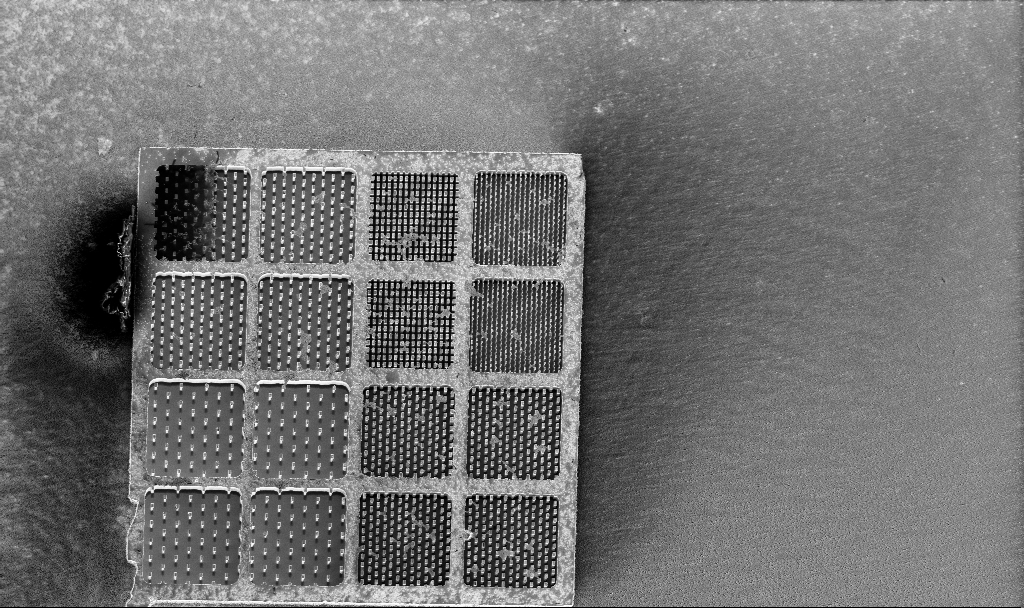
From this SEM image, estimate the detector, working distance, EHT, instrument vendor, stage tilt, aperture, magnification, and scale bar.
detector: InLens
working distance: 3.3 mm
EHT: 5 kV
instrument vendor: Zeiss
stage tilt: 8°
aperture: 30 µm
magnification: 0.659 K X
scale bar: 100000 nm